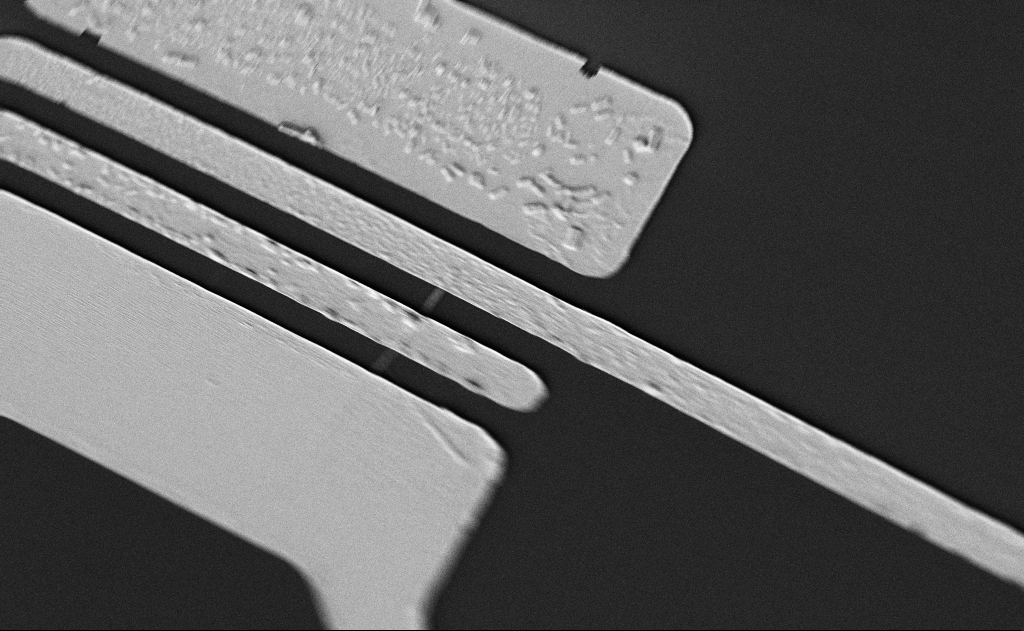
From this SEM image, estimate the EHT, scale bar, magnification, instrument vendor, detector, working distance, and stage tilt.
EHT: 5 kV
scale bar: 2000 nm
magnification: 8 K X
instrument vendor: Zeiss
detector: SE2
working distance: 21 mm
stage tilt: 0°